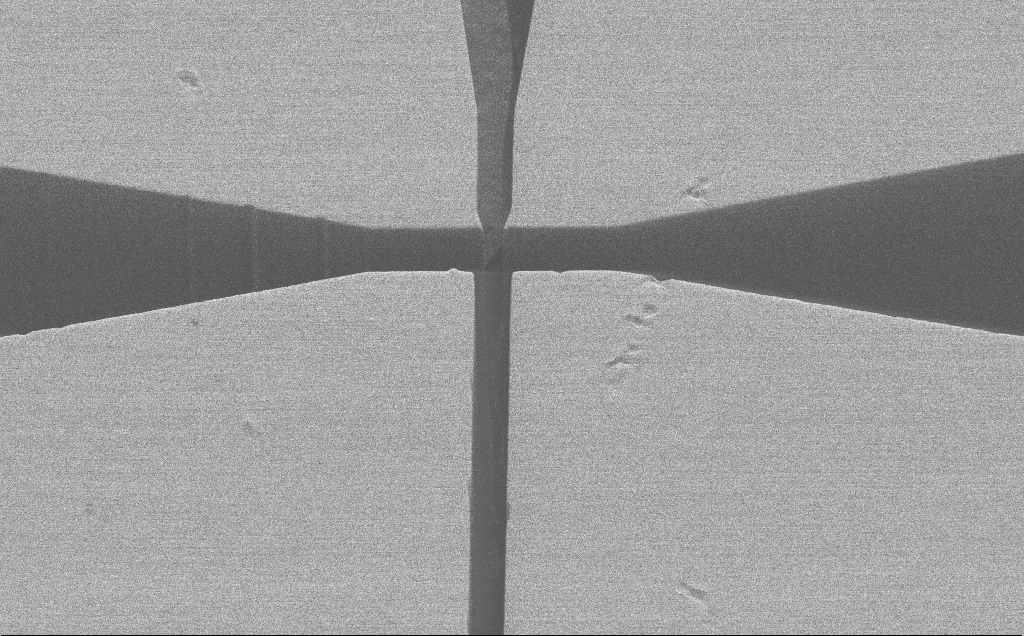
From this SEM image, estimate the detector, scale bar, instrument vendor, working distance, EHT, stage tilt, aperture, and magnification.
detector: SE2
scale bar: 20000 nm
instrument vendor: Zeiss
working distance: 6 mm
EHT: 1.2 kV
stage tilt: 45°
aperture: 30 µm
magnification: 1.17 K X